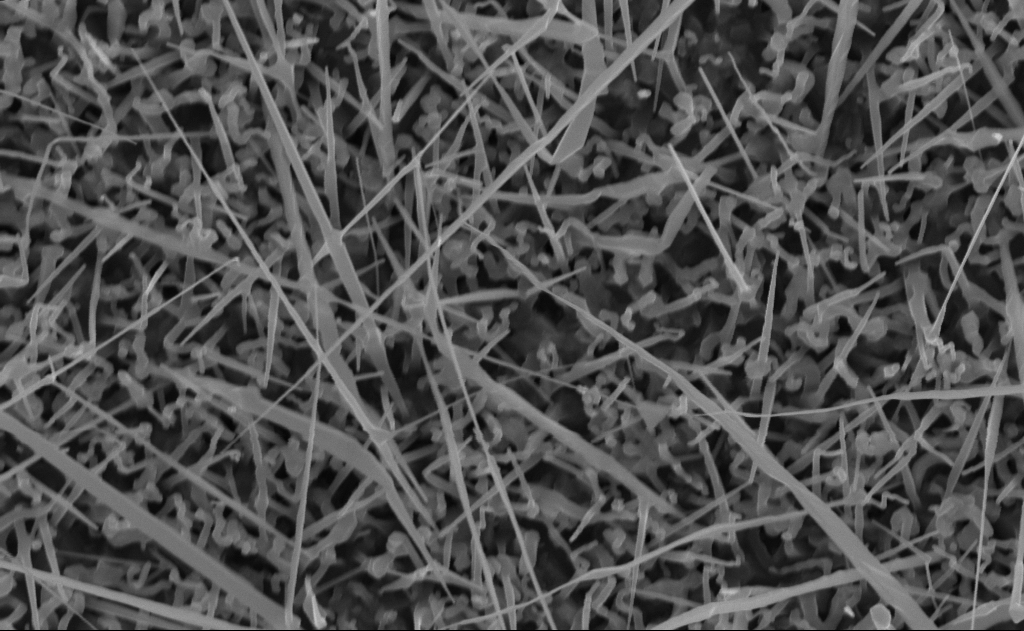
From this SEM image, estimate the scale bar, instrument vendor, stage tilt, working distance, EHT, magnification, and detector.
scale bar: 1000 nm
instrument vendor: Zeiss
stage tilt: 0°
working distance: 10 mm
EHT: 10 kV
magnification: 40 K X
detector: InLens